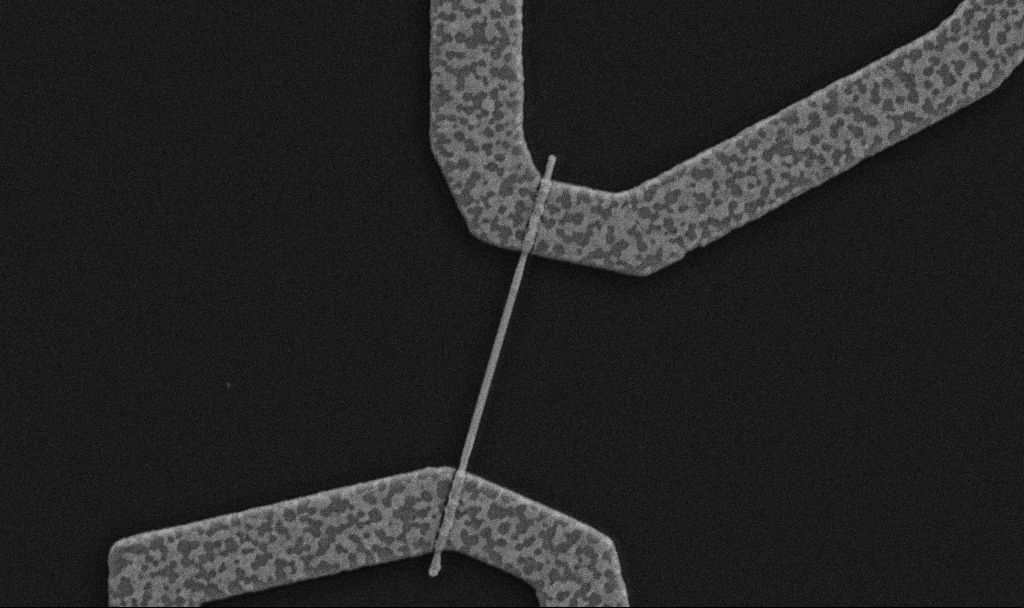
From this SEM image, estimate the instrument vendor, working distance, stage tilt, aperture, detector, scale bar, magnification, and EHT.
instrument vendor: Zeiss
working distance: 10.7 mm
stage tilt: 0°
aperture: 30 µm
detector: SE2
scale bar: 1000 nm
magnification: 30 K X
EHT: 5 kV